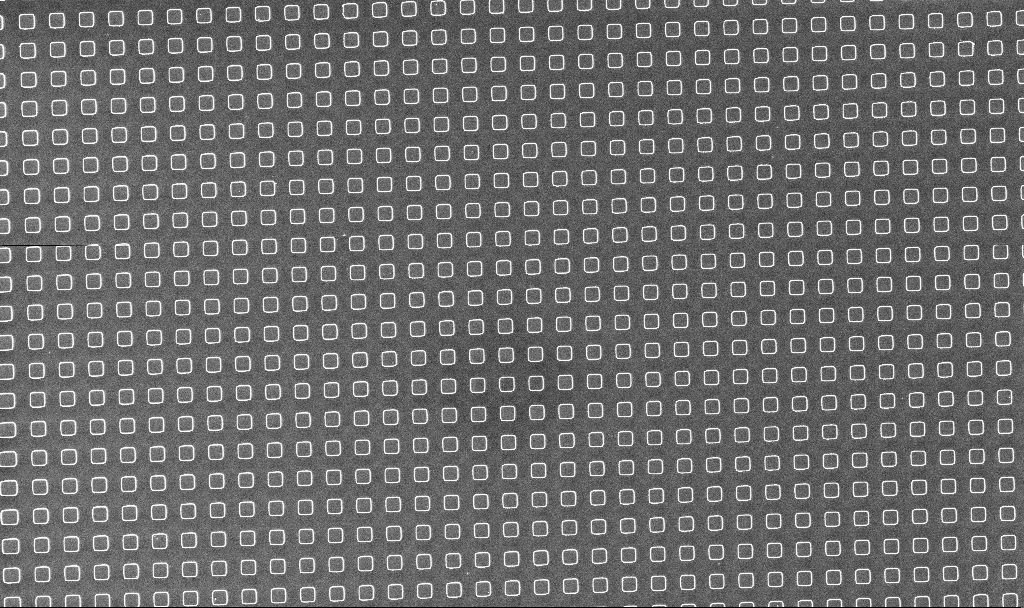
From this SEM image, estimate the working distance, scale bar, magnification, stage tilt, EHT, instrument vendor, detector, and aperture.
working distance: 5.2 mm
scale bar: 10000 nm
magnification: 1.35 K X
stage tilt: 0°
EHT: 5 kV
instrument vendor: Zeiss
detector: InLens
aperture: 30 µm